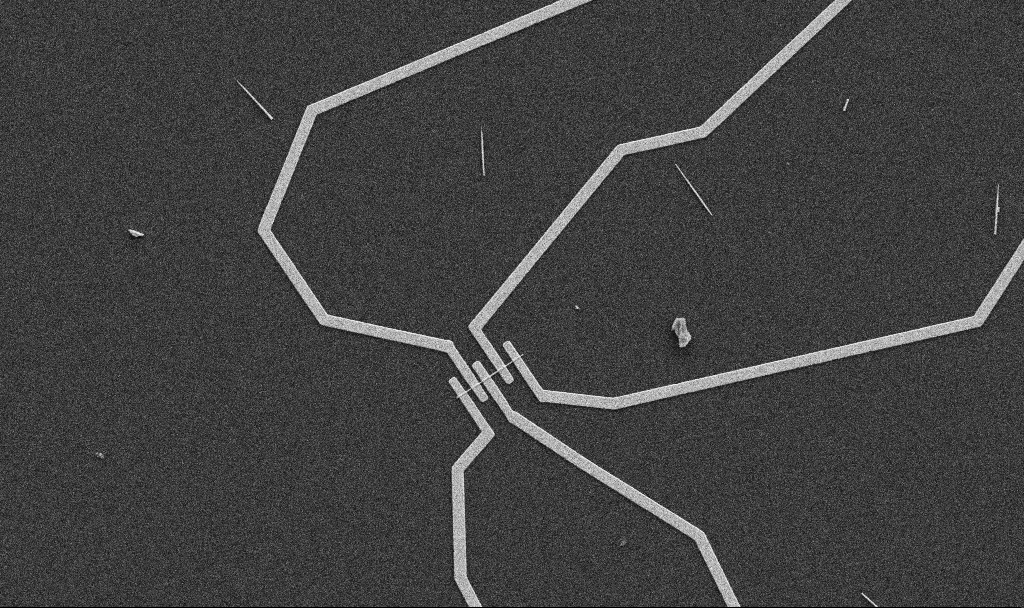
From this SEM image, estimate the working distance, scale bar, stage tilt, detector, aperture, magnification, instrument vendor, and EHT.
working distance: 10.7 mm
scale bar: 10000 nm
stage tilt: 0°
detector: SE2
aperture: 30 µm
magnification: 5 K X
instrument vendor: Zeiss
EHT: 5 kV